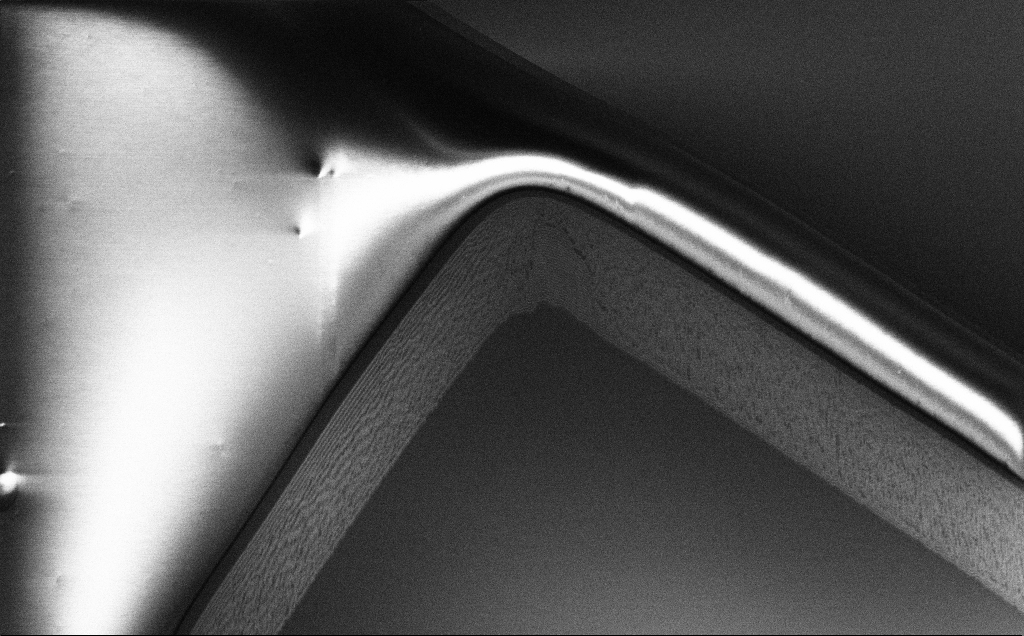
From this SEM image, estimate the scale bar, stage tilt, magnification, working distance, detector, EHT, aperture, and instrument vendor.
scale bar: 20000 nm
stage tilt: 45°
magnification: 2.82 K X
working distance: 7 mm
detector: InLens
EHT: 1.2 kV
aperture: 30 µm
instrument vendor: Zeiss